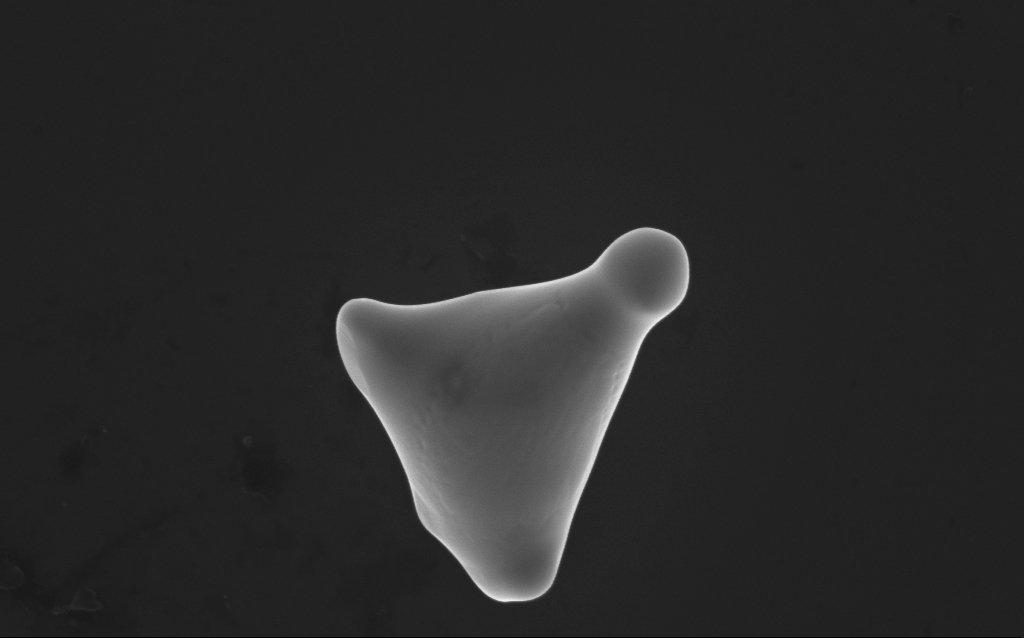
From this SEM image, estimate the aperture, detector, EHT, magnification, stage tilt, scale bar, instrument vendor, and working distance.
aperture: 30 µm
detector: InLens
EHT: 10 kV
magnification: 46.26 K X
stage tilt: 0°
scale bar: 1000 nm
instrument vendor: Zeiss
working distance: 4 mm